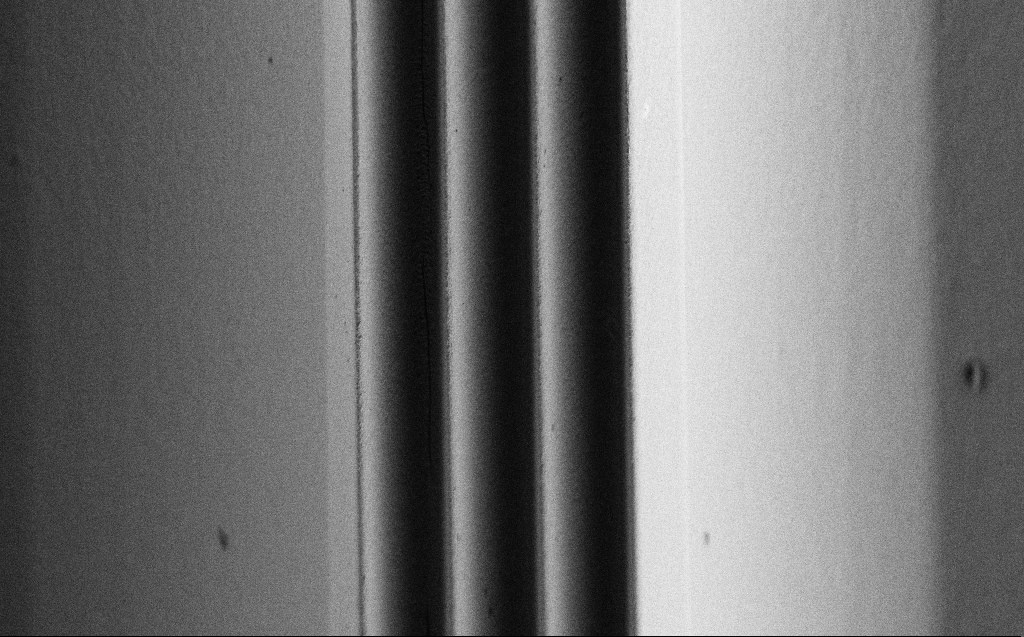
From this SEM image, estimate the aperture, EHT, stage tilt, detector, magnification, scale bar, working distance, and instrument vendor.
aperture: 30 µm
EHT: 1 kV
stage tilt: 44.6°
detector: SE2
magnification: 2.95 K X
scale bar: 10000 nm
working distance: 5 mm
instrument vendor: Zeiss